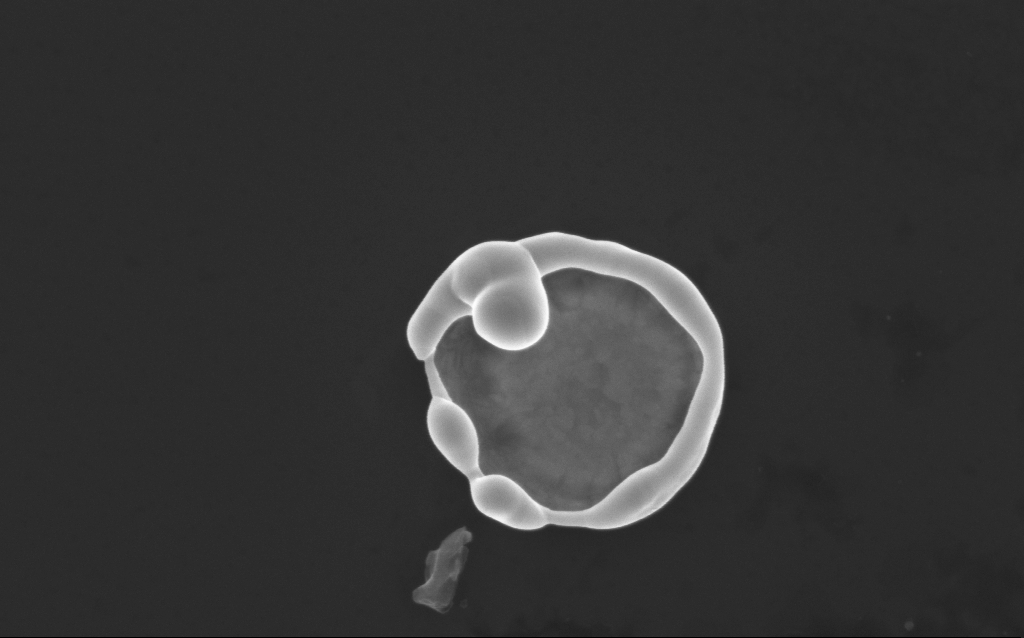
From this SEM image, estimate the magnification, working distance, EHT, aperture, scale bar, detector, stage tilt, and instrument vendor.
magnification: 60.82 K X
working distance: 4 mm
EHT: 10 kV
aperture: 30 µm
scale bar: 1000 nm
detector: InLens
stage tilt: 0°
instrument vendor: Zeiss